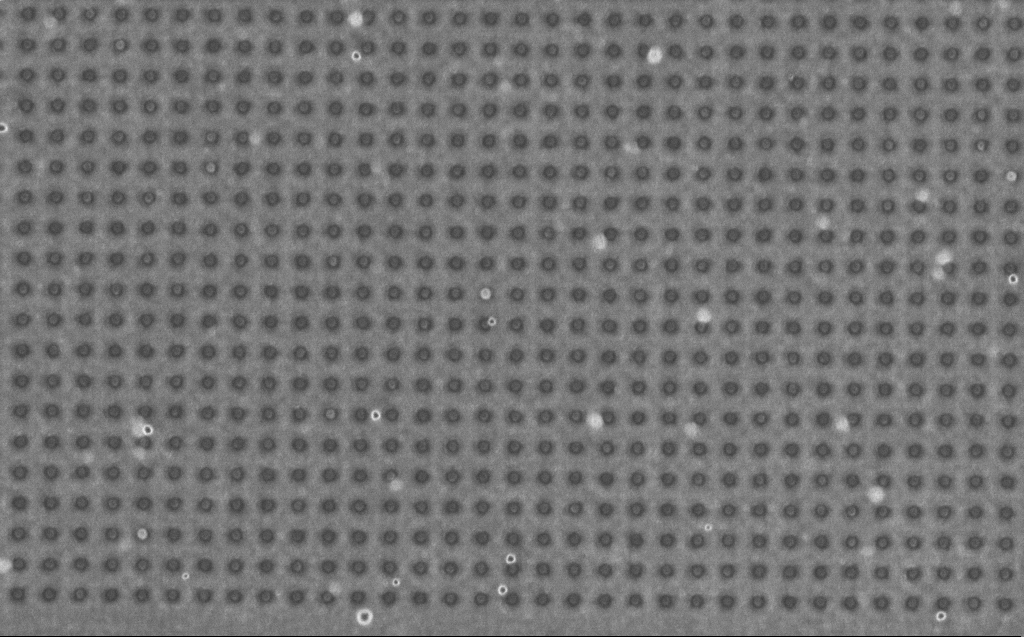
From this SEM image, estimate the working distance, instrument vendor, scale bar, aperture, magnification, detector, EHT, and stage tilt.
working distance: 4 mm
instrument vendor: Zeiss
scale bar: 2000 nm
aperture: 30 µm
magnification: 28.35 K X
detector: InLens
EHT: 1 kV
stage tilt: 0°